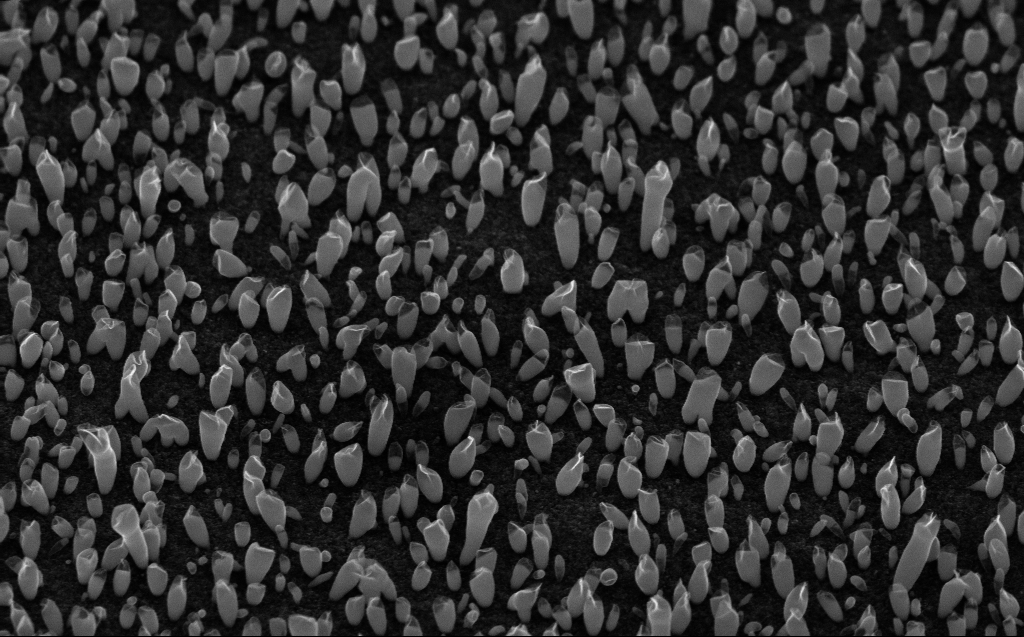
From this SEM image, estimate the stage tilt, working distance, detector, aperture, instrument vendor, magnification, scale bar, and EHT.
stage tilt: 45°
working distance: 5 mm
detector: InLens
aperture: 30 µm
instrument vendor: Zeiss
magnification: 50 K X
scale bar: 1000 nm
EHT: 10 kV